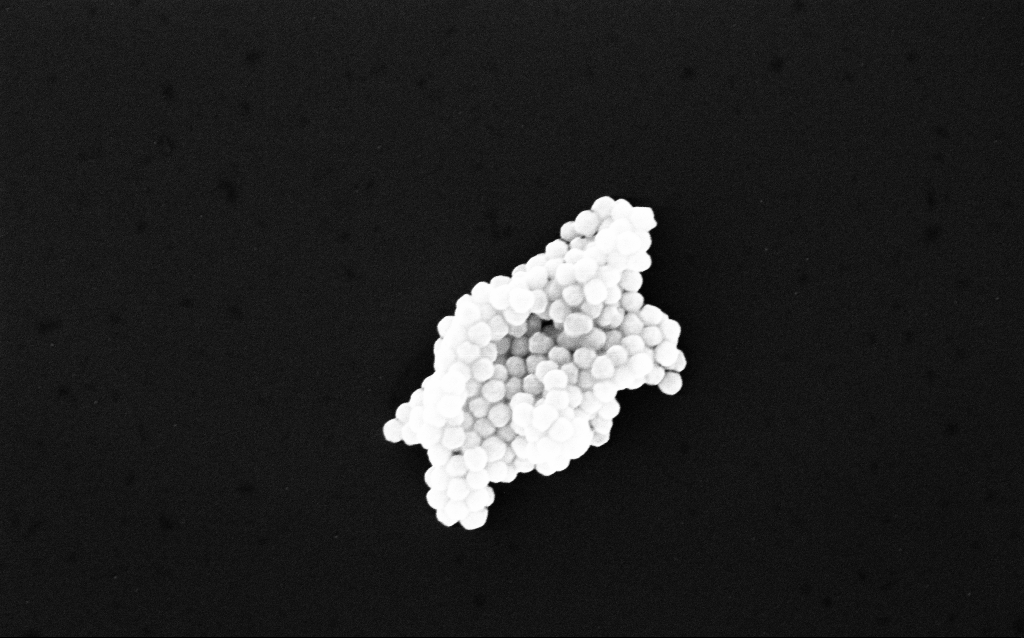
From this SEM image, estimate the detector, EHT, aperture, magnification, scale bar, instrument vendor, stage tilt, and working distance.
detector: InLens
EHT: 10 kV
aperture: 30 µm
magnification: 139.01 K X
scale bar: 200 nm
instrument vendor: Zeiss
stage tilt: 0°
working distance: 3.1 mm